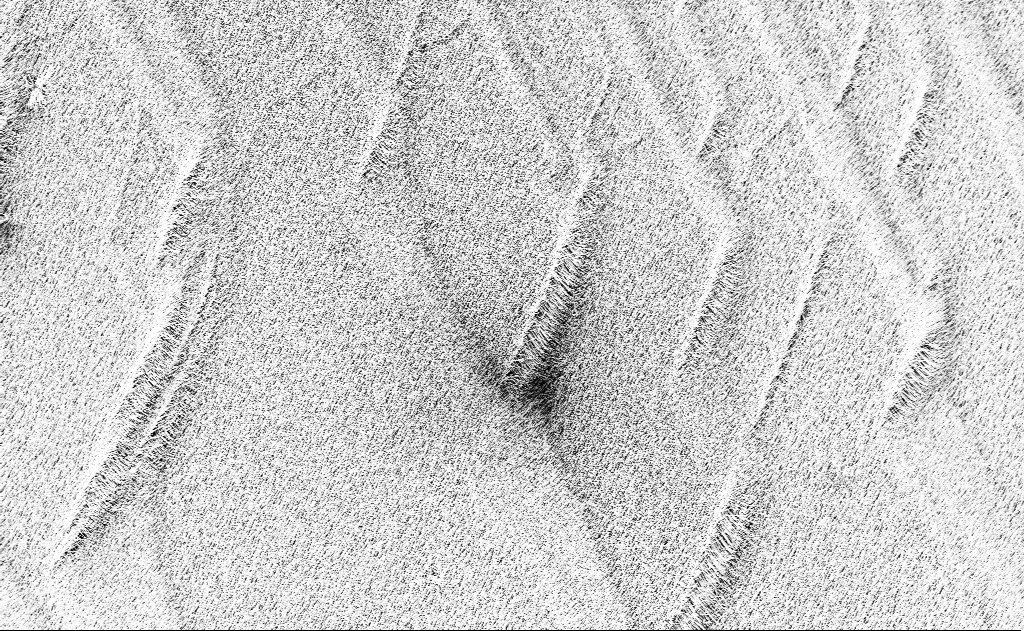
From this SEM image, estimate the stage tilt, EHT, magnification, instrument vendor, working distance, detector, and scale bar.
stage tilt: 0°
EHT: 10 kV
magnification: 1.95 K X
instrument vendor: Zeiss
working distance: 13 mm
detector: InLens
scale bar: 20000 nm